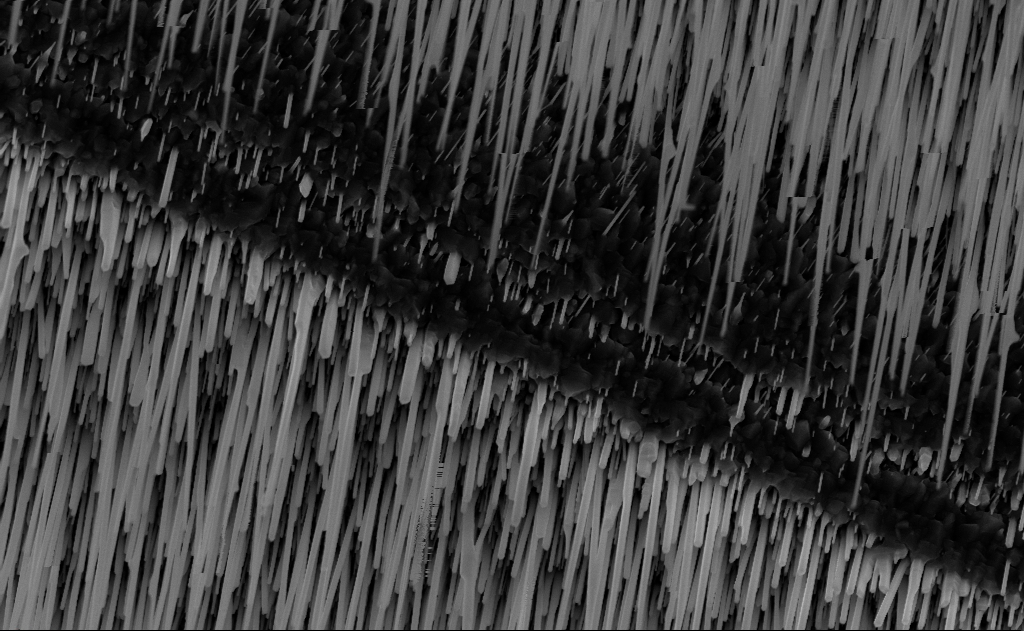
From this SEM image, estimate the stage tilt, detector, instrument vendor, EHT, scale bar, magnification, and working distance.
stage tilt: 0°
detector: InLens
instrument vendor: Zeiss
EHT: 10 kV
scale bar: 2000 nm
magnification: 20 K X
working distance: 9 mm